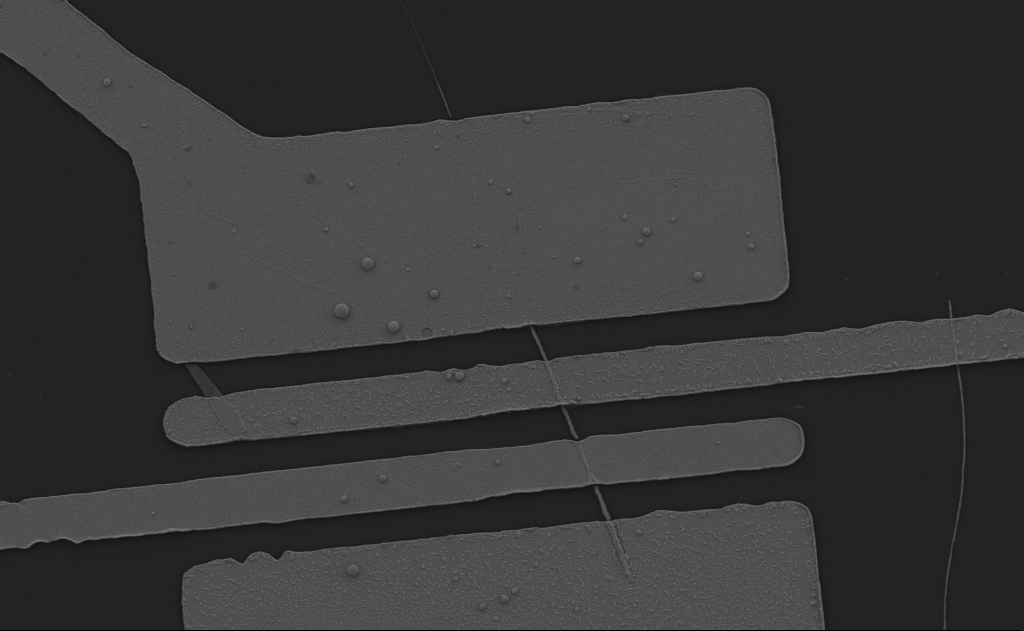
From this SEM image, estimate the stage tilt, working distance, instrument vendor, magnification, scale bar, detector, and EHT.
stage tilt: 0°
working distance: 13 mm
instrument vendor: Zeiss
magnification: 7.7 K X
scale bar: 2000 nm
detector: SE2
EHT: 5 kV